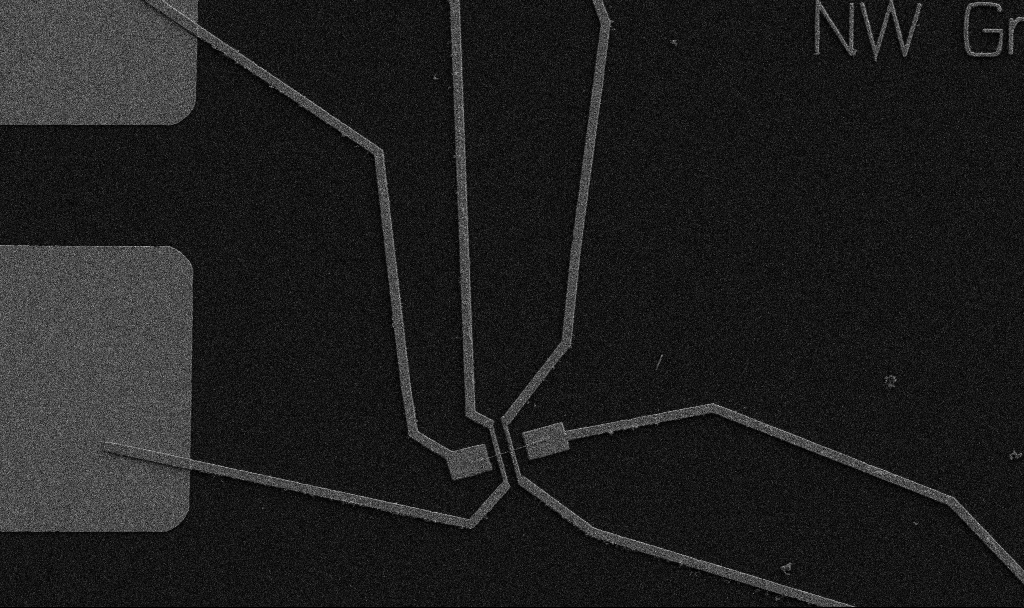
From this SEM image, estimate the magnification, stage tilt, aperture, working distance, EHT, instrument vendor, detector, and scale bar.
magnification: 5 K X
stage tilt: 0°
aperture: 30 µm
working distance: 10.7 mm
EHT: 5 kV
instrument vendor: Zeiss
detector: SE2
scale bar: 10000 nm